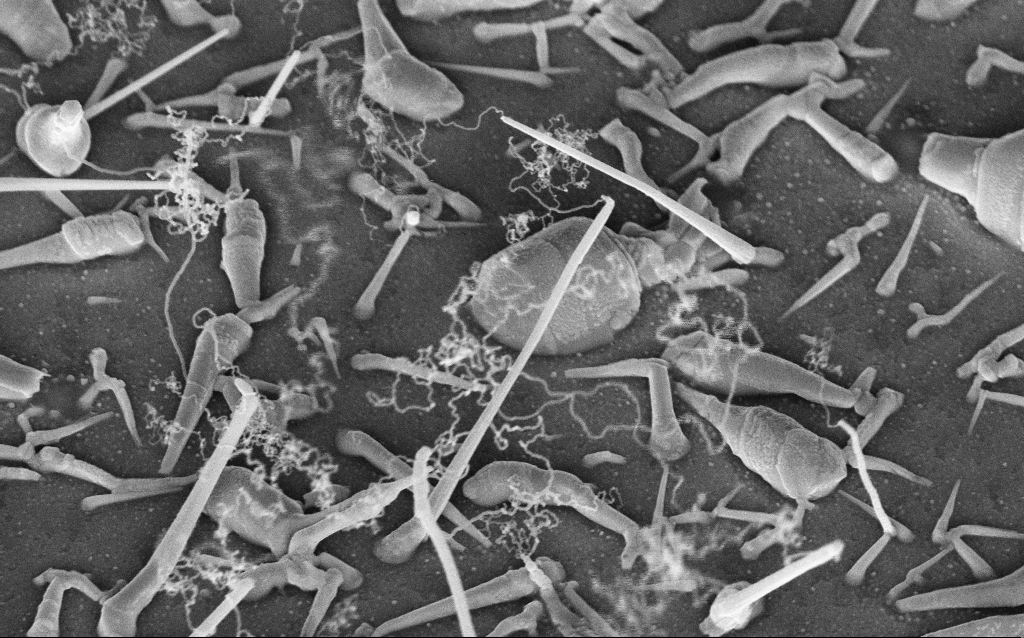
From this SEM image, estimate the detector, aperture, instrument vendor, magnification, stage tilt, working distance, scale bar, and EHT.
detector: InLens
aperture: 30 µm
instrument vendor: Zeiss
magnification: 45.79 K X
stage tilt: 42°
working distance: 8 mm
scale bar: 1000 nm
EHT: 5 kV